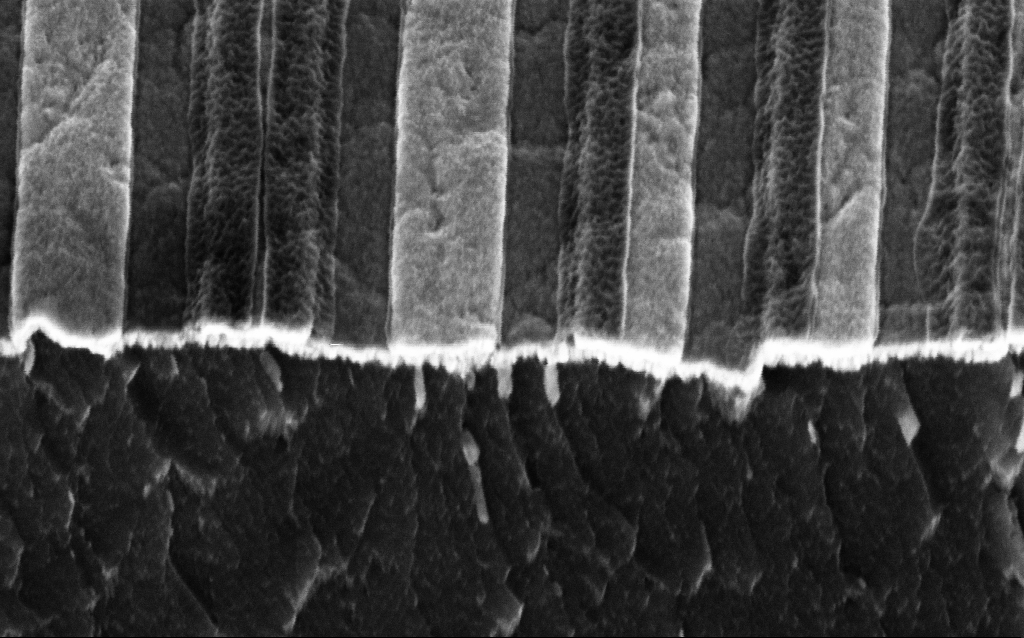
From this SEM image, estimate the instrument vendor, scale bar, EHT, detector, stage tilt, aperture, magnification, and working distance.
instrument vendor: Zeiss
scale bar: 200 nm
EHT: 3 kV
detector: InLens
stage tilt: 45°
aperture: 30 µm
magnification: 139.44 K X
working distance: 6 mm